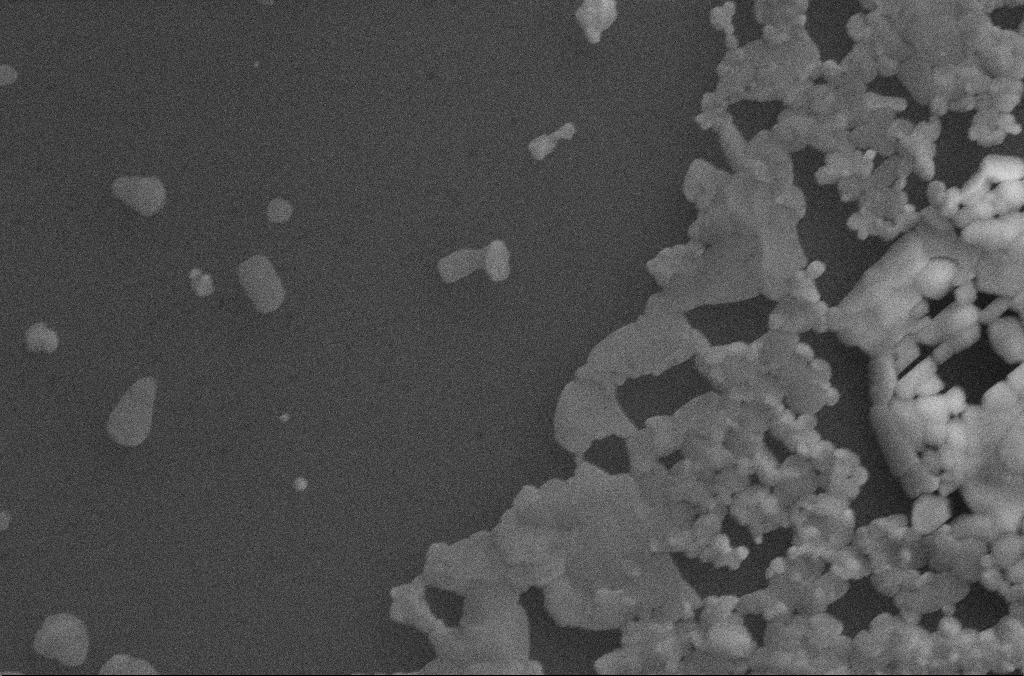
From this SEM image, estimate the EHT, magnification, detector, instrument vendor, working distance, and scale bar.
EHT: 20 kV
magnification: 10 K X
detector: SE2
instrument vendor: Zeiss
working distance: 2.8 mm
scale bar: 2000 nm